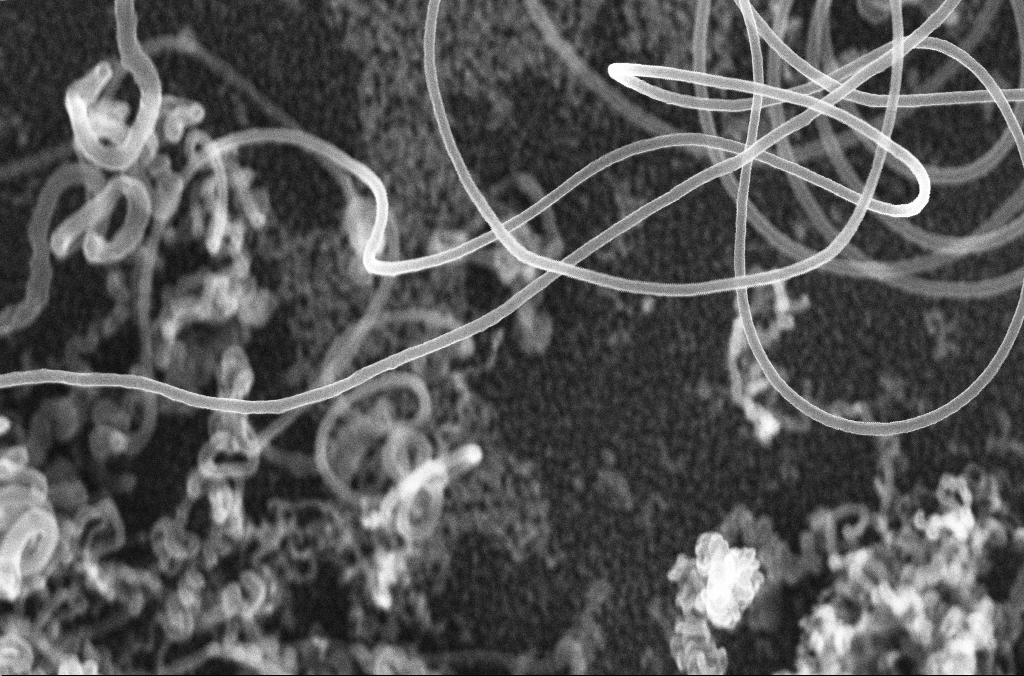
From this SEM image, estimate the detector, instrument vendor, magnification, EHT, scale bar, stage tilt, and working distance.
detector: InLens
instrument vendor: Zeiss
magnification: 40.56 K X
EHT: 20 kV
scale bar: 1000 nm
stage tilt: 0°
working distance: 4.2 mm